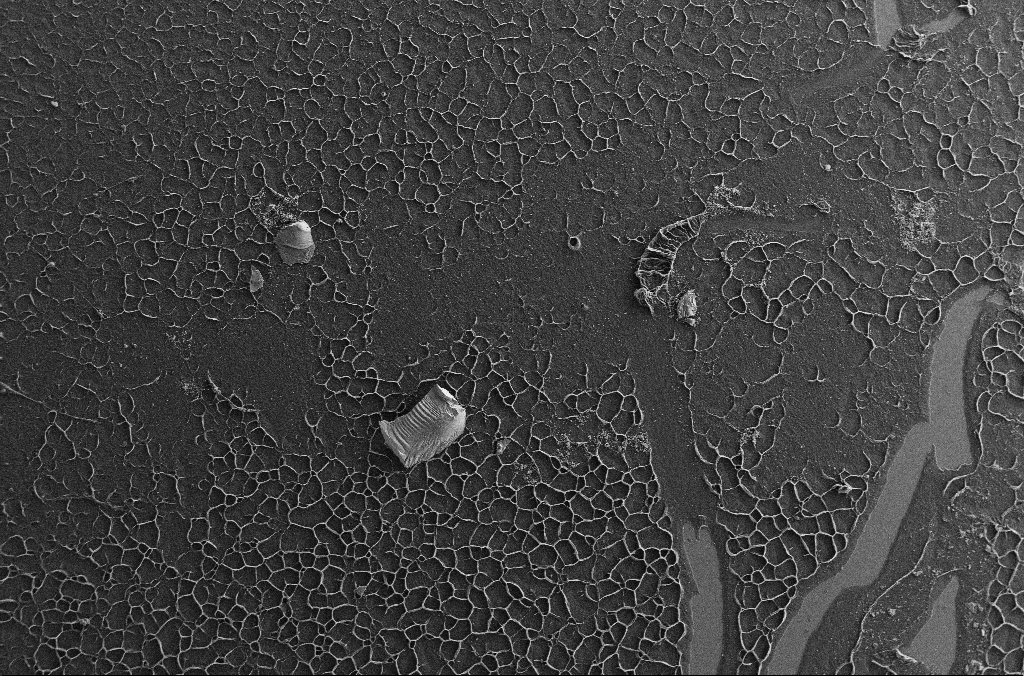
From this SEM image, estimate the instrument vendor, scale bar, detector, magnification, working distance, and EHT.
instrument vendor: Zeiss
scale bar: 100000 nm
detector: SE2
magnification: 0.15 K X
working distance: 2.9 mm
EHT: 7 kV